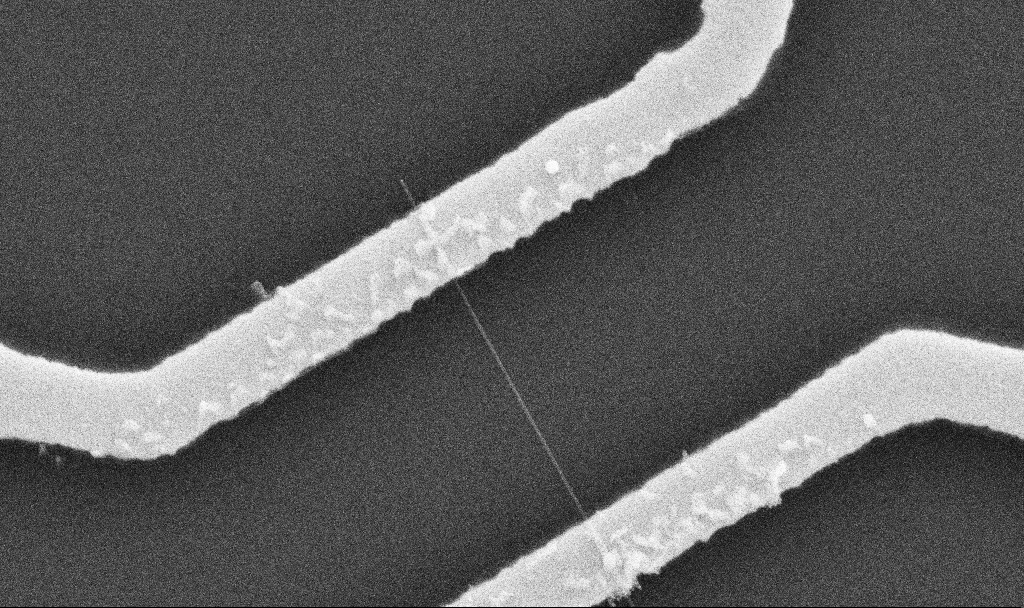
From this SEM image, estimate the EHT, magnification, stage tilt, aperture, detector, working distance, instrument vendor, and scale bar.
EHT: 10 kV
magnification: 50 K X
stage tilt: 0°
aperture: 30 µm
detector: SE2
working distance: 10.7 mm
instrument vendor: Zeiss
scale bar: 1000 nm